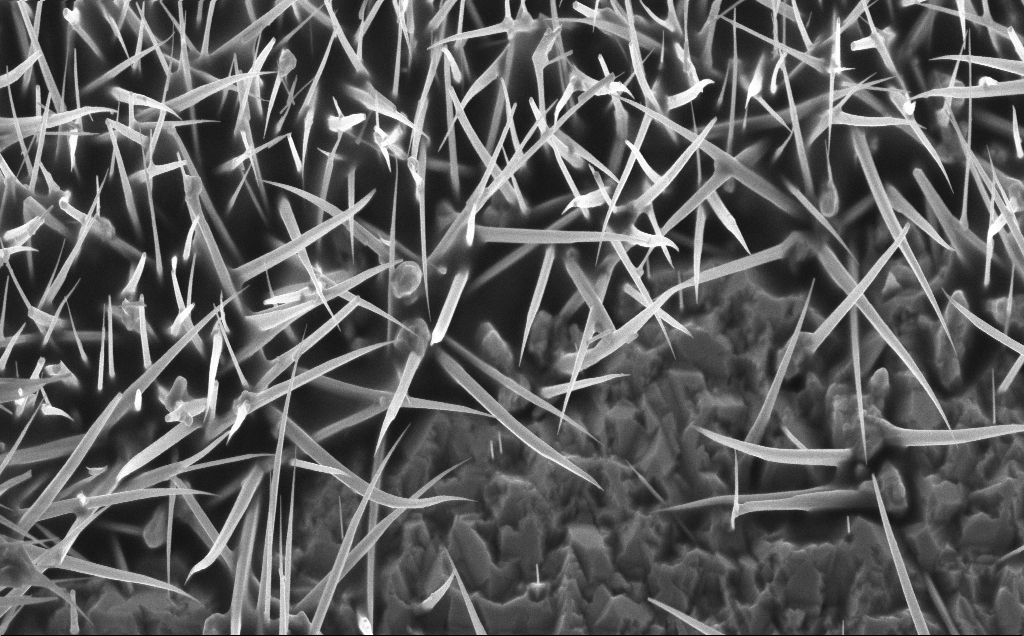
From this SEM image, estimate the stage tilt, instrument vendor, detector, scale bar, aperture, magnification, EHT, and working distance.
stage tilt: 30°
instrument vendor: Zeiss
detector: InLens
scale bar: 2000 nm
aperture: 30 µm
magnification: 18.94 K X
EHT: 5 kV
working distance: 7 mm